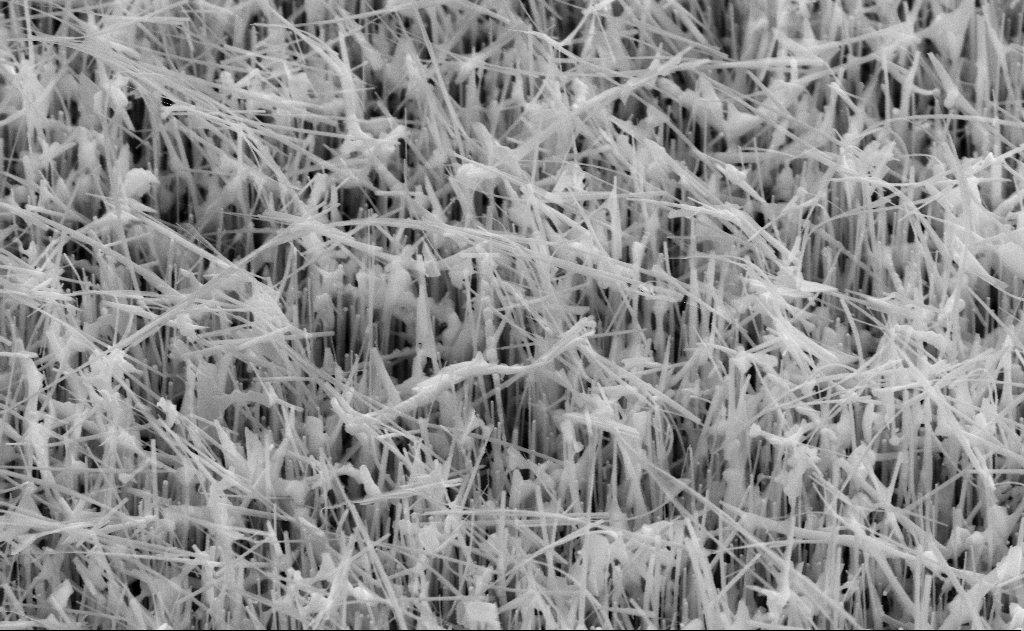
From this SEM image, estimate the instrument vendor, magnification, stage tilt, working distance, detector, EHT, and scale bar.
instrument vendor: Zeiss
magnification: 40 K X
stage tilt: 45°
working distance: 14 mm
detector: SE2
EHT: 10 kV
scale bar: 1000 nm